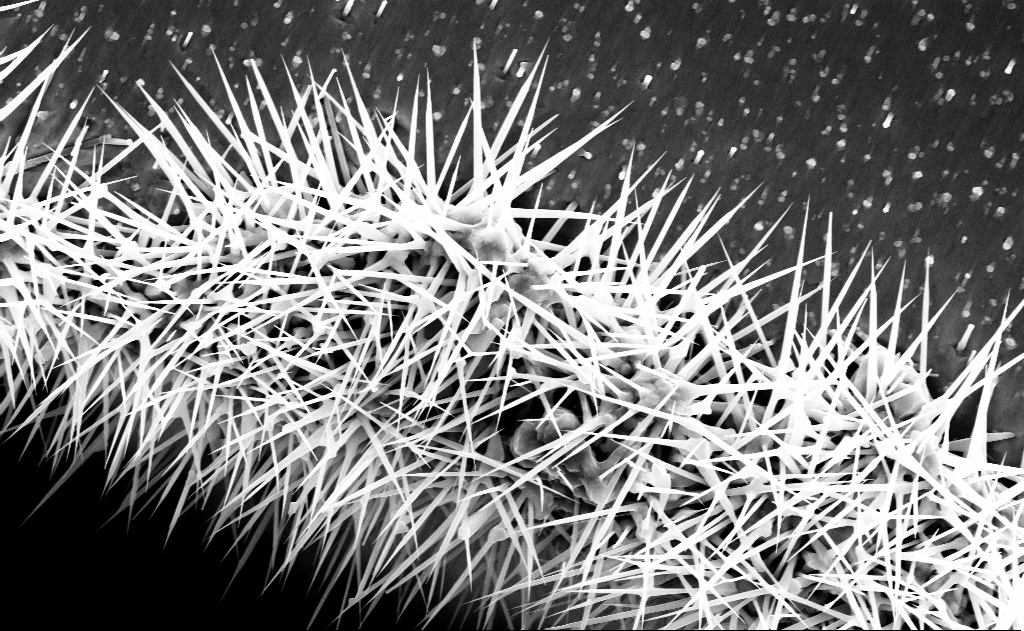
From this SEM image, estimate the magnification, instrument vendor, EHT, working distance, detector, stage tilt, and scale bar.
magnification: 20 K X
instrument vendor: Zeiss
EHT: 10 kV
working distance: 9 mm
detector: InLens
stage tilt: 0°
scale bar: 2000 nm